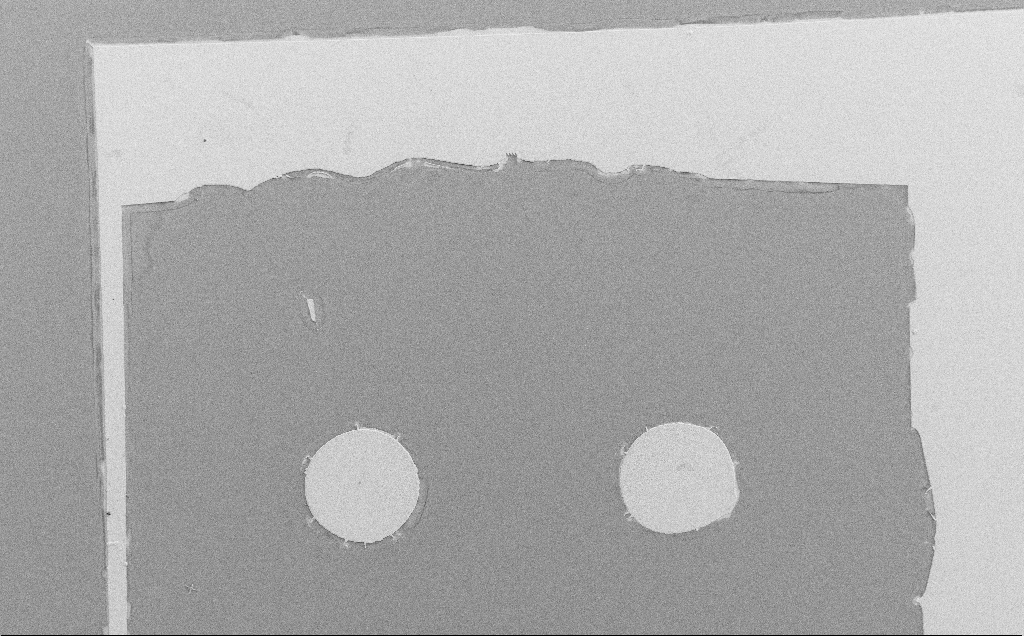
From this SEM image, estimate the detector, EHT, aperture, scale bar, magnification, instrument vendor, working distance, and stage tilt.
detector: SE2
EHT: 10 kV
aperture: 30 µm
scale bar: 200000 nm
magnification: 0.098 K X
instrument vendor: Zeiss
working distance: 7 mm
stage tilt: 0°